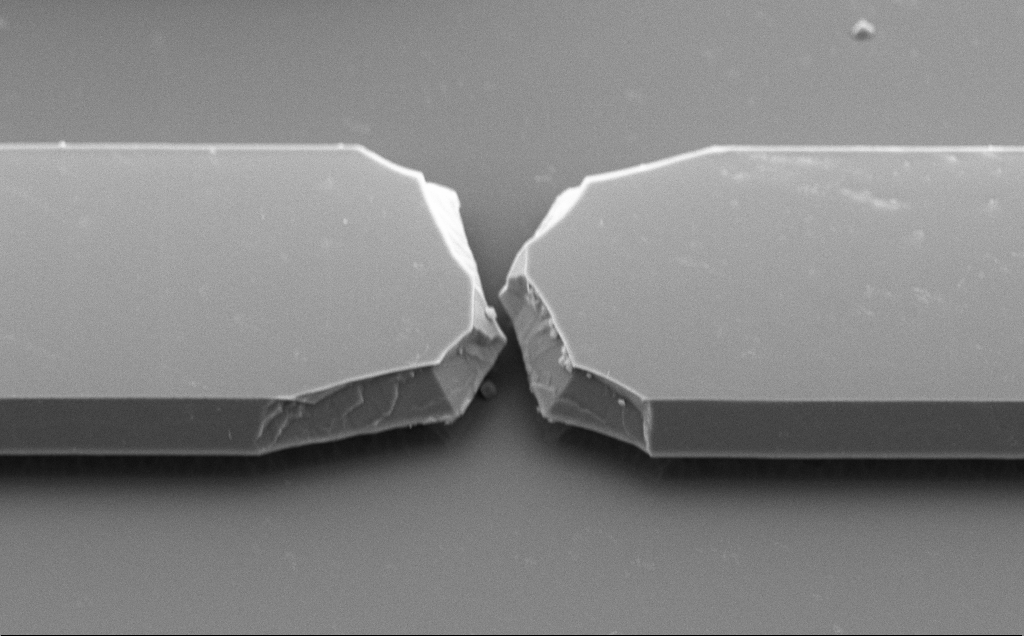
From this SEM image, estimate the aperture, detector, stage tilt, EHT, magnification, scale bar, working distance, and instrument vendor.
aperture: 30 µm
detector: SE2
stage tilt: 50°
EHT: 5 kV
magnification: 7.41 K X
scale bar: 2000 nm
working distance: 10 mm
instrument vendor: Zeiss